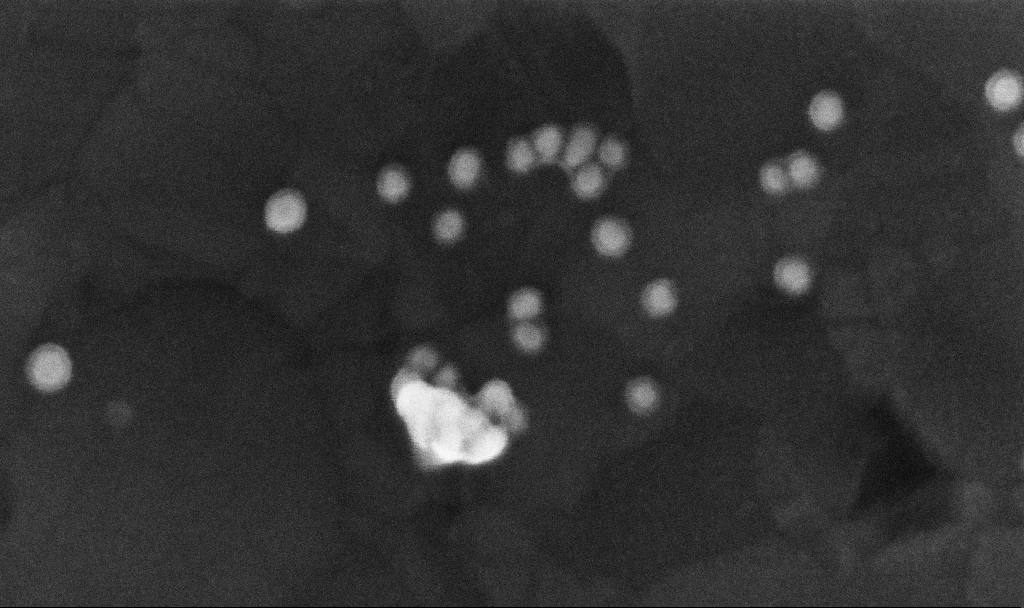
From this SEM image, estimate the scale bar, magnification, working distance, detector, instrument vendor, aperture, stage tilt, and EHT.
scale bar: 100 nm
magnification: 634.23 K X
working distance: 3.7 mm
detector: InLens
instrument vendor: Zeiss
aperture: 30 µm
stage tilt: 0°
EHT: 10 kV